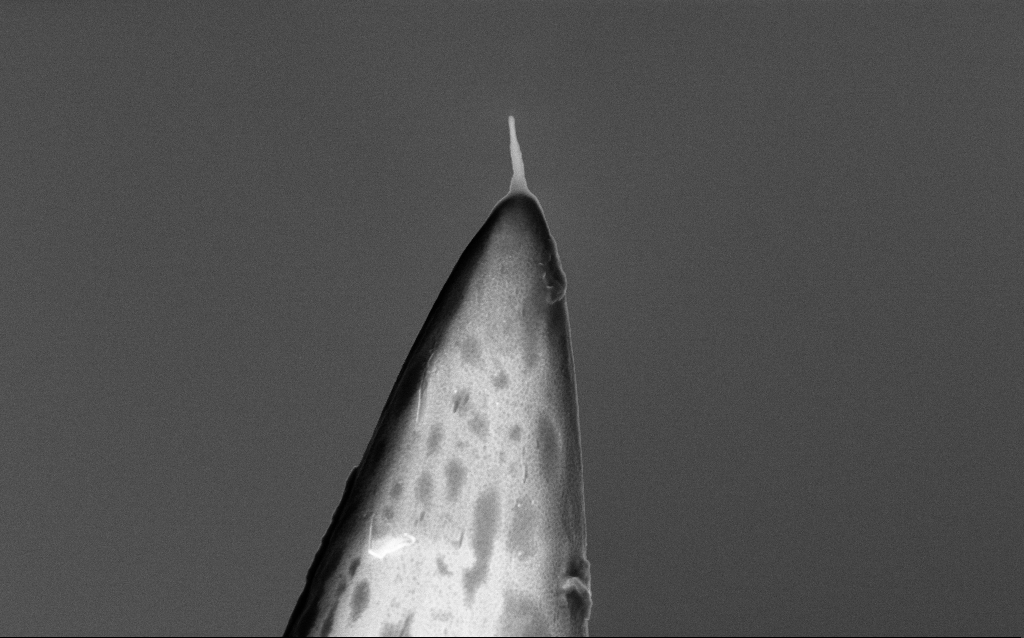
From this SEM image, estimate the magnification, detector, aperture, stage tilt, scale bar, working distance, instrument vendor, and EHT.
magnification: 43.99 K X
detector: InLens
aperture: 30 µm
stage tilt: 0°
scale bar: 1000 nm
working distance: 3 mm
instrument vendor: Zeiss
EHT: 5 kV